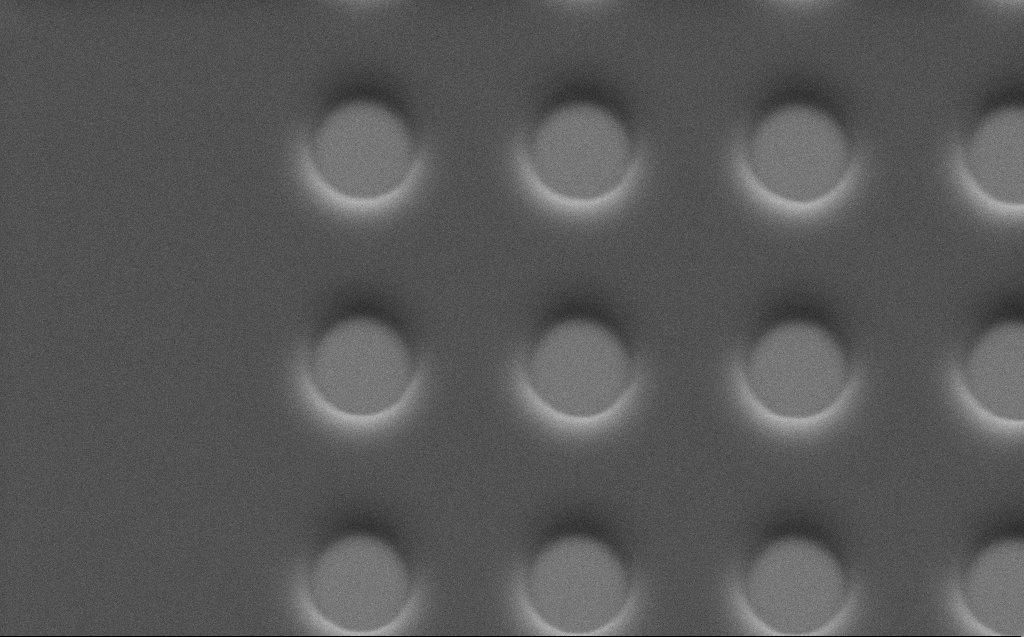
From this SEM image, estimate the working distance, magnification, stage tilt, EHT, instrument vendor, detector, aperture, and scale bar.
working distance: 5 mm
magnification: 20.3 K X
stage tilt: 45°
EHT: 5 kV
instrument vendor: Zeiss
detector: SE2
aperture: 30 µm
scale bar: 2000 nm